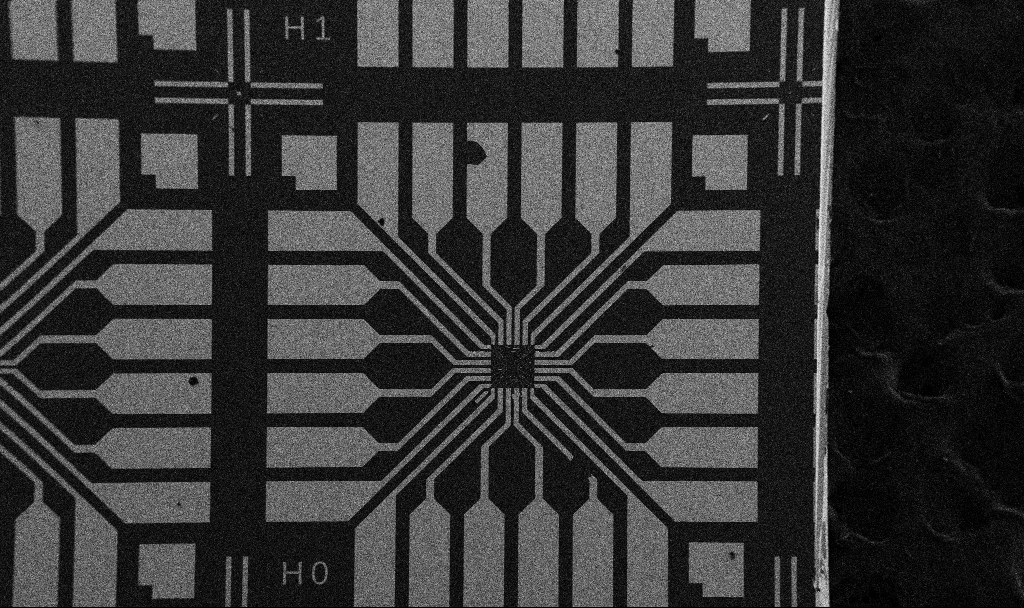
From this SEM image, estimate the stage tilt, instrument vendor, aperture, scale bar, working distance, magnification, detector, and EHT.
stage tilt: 0°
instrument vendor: Zeiss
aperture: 30 µm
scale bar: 200000 nm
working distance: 10.7 mm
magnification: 0.1 K X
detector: SE2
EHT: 5 kV